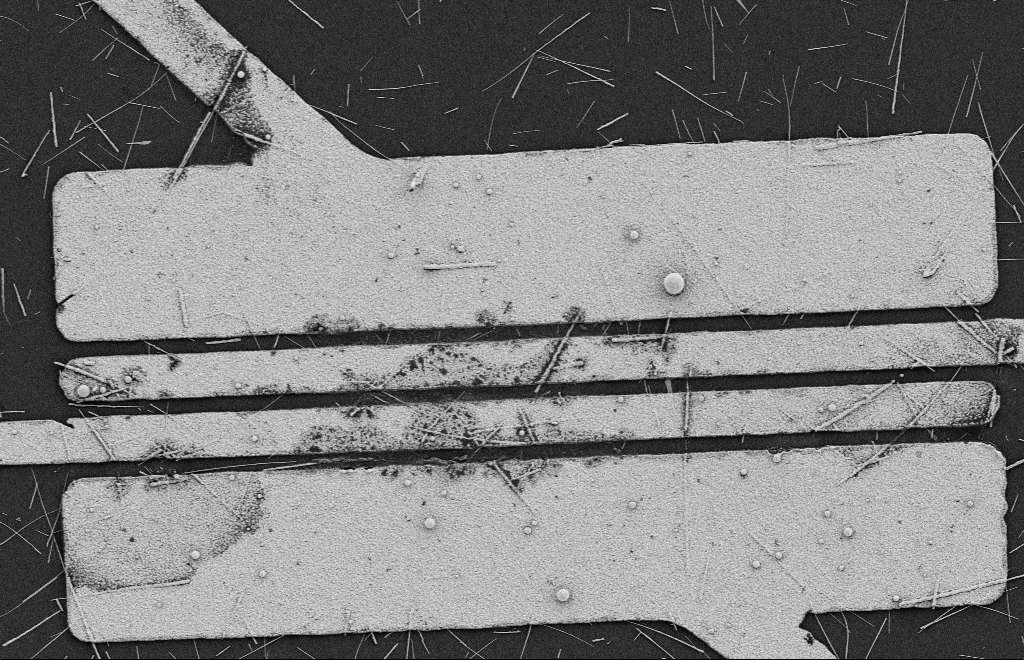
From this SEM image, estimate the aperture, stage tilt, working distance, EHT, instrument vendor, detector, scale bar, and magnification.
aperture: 20 µm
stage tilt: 0°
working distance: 9 mm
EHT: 2 kV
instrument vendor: Zeiss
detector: SE2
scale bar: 2000 nm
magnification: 5.6 K X